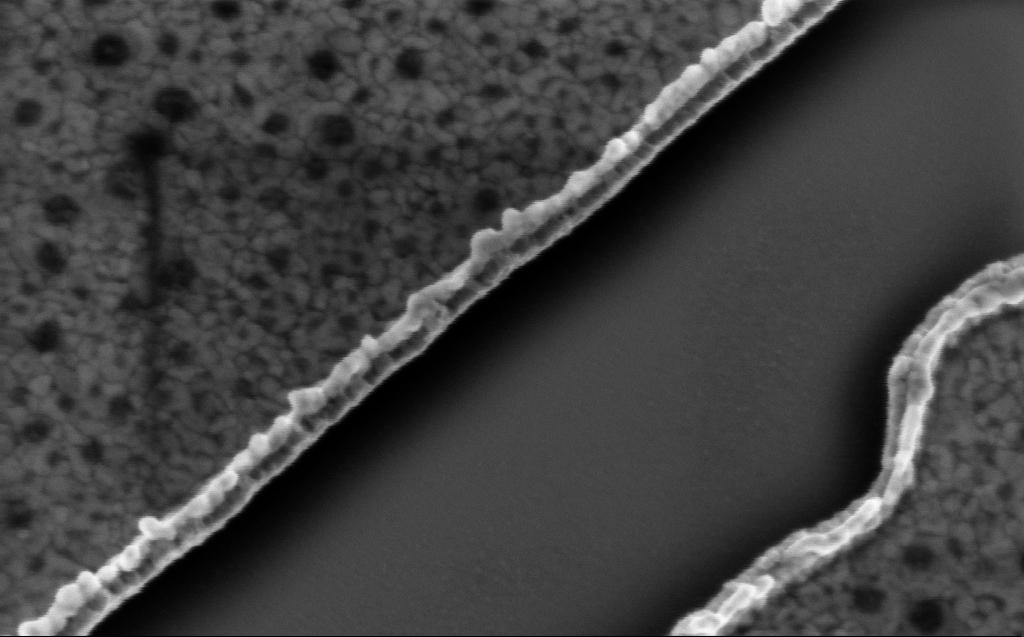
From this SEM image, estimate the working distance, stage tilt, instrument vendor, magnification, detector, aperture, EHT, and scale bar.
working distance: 4 mm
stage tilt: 20°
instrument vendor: Zeiss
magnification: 159.36 K X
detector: InLens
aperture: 30 µm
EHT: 3 kV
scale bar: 200 nm